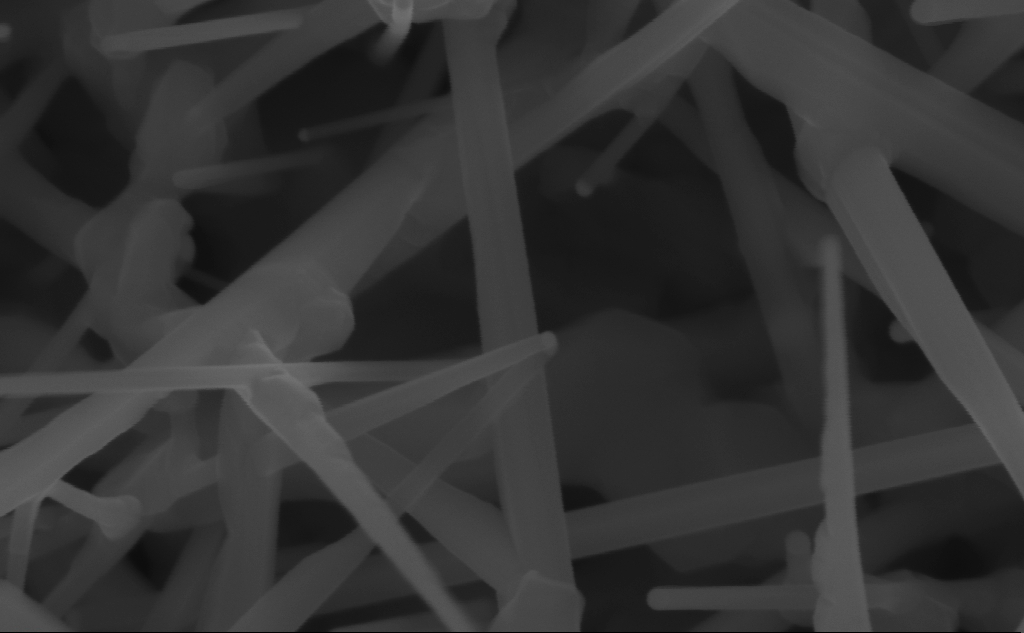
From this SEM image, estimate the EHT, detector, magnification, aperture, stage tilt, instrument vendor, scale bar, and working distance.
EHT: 10 kV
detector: InLens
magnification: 235.38 K X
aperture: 30 µm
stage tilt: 0°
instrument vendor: Zeiss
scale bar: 200 nm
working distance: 7 mm